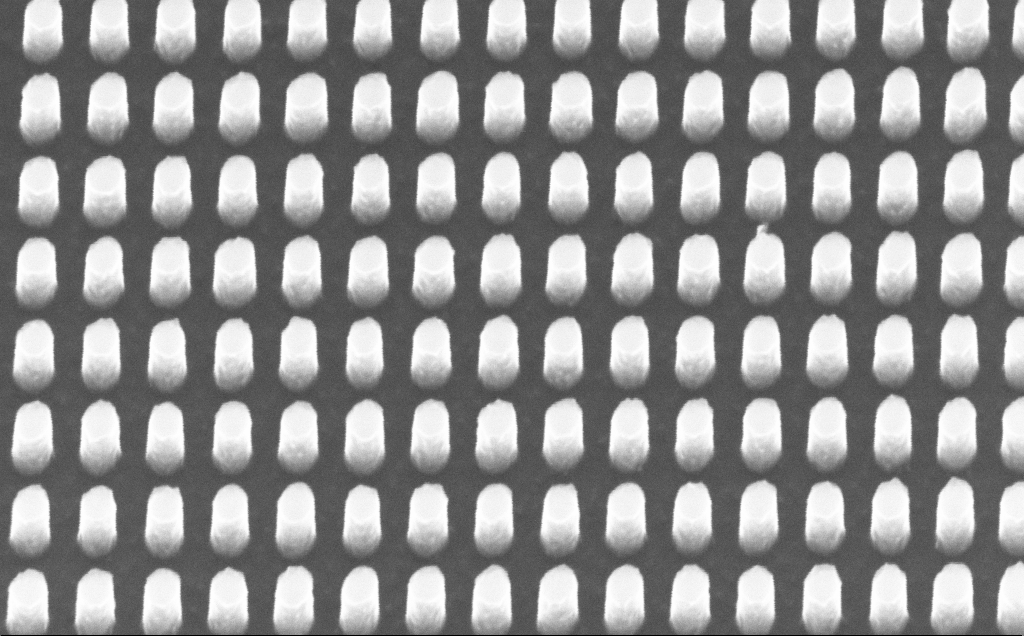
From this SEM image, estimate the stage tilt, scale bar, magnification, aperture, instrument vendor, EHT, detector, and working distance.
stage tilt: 30°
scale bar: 200 nm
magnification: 121.38 K X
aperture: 30 µm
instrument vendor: Zeiss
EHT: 10 kV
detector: InLens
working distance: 6 mm